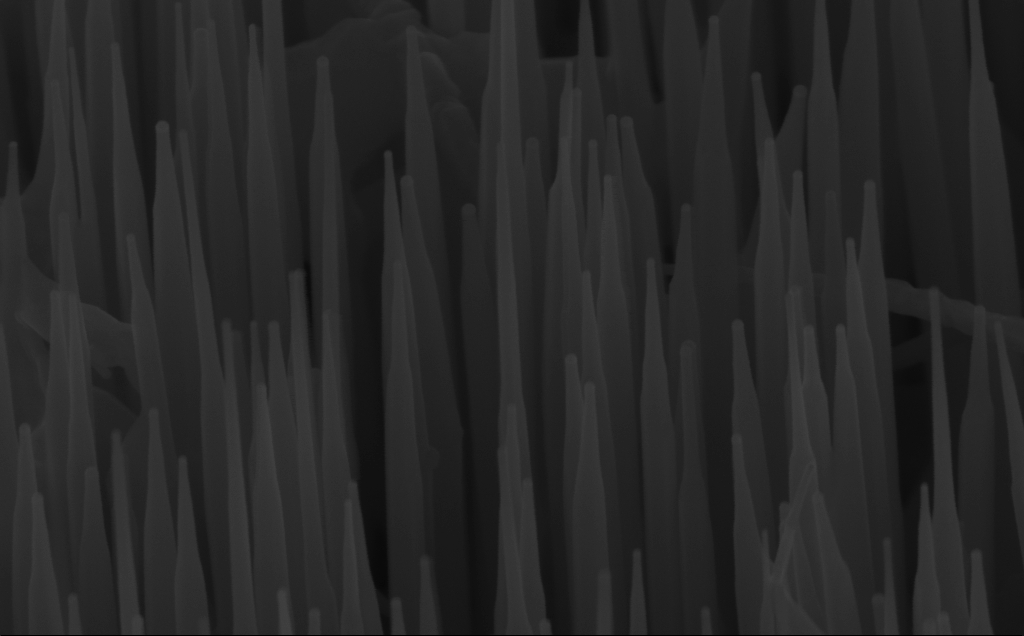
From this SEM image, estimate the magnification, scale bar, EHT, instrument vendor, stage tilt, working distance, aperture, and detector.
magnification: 150 K X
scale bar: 200 nm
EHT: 10 kV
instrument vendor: Zeiss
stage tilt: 45°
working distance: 6 mm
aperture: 30 µm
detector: InLens